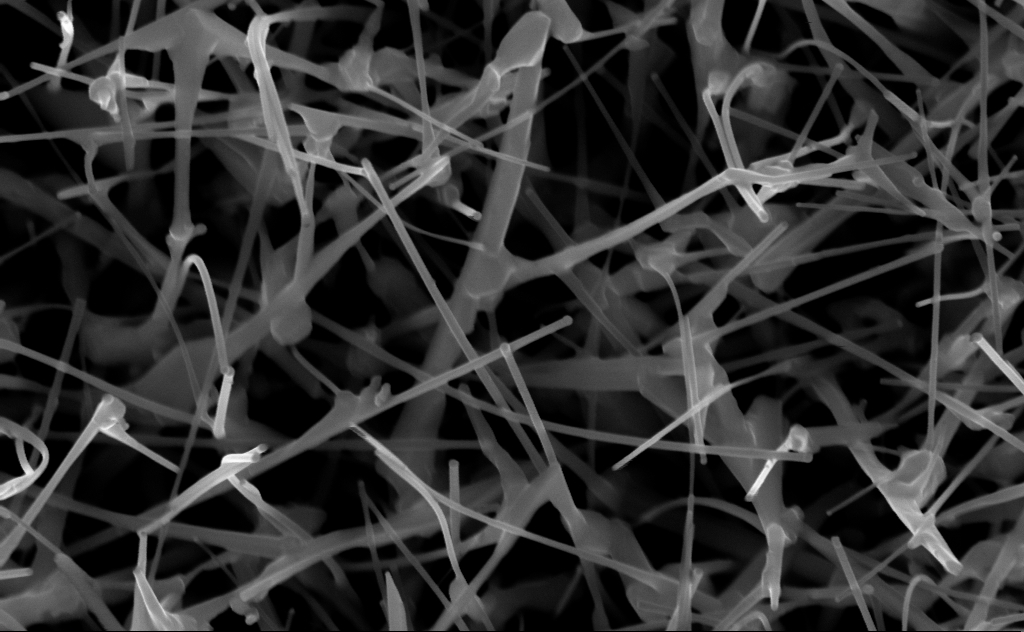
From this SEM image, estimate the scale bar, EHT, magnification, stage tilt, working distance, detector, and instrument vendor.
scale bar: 200 nm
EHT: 10 kV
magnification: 80 K X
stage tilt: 0°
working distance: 6 mm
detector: InLens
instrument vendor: Zeiss